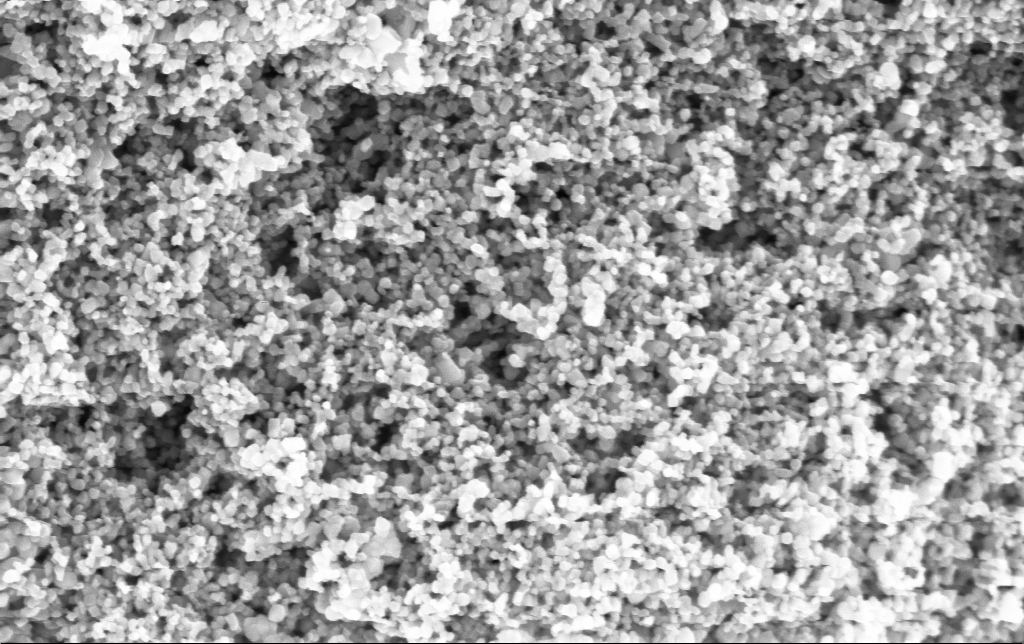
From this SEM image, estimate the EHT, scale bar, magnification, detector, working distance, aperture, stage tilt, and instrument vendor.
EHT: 5 kV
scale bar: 100 nm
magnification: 135 K X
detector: InLens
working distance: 4.9 mm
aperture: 30 µm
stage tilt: -0°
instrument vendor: Zeiss